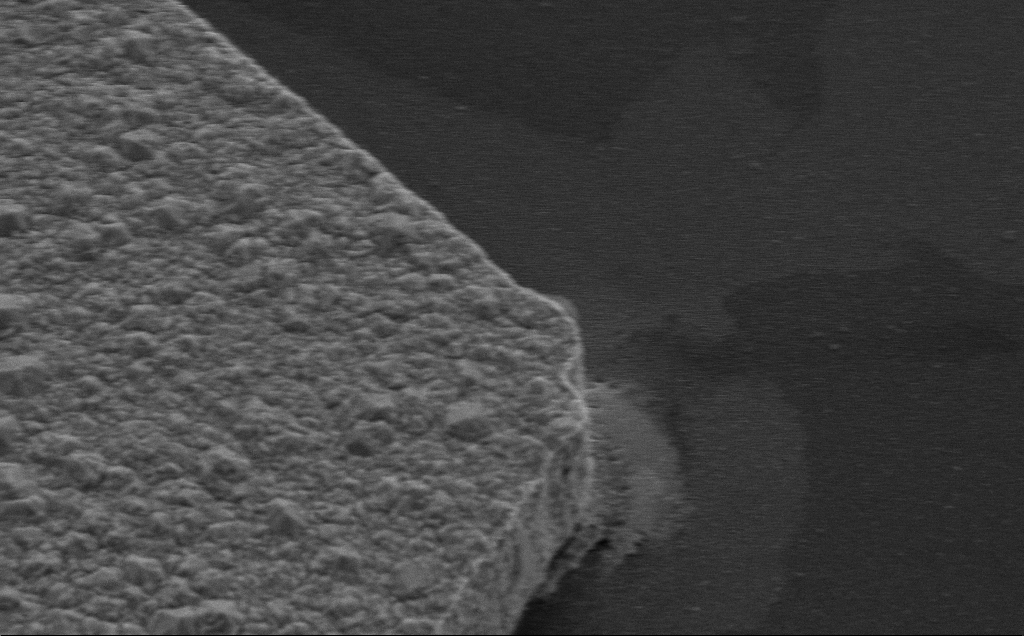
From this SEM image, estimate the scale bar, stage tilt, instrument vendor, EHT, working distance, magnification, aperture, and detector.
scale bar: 2000 nm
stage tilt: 35°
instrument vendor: Zeiss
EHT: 10 kV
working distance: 8 mm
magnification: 15.71 K X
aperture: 30 µm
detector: SE2